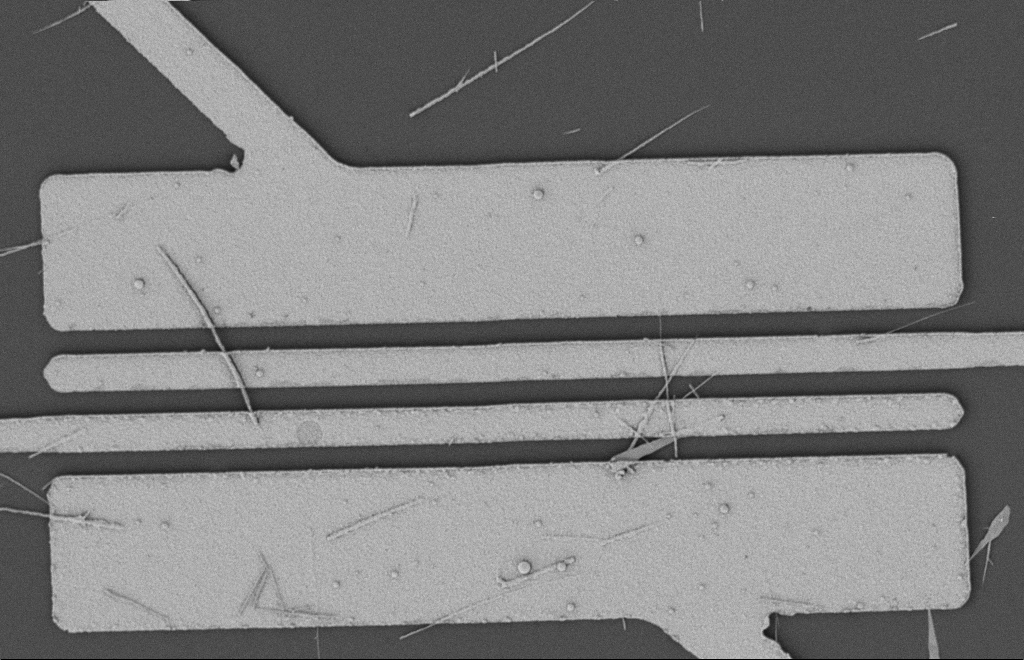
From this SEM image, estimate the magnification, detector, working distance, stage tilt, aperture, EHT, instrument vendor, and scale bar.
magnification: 5.5 K X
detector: SE2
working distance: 12 mm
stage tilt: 0°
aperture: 20 µm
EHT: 2 kV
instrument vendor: Zeiss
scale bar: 2000 nm